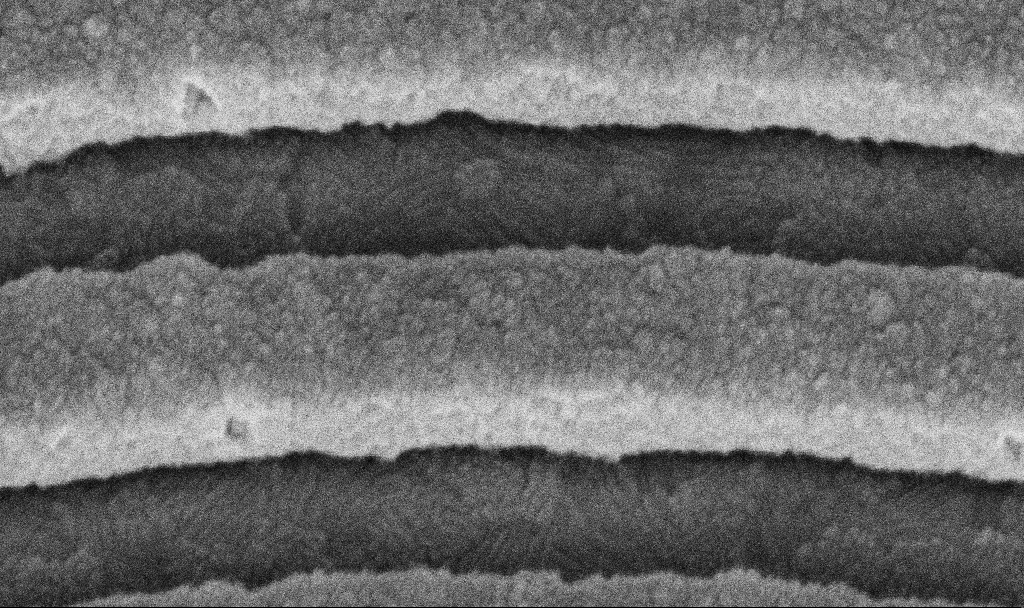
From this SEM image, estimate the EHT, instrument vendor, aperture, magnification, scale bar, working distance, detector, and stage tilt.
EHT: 5 kV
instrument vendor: Zeiss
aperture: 30 µm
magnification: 196.76 K X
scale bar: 200 nm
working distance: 8.7 mm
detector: InLens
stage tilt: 45°